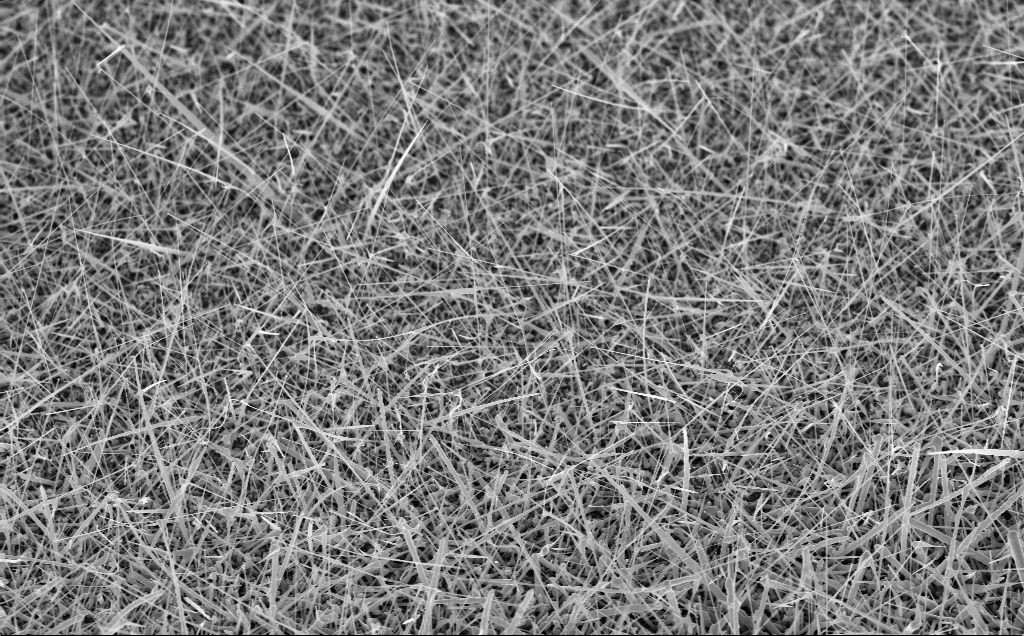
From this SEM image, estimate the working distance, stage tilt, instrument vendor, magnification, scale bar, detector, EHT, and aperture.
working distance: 8 mm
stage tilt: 45°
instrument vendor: Zeiss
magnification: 10 K X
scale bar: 2000 nm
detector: InLens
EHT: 10 kV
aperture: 30 µm